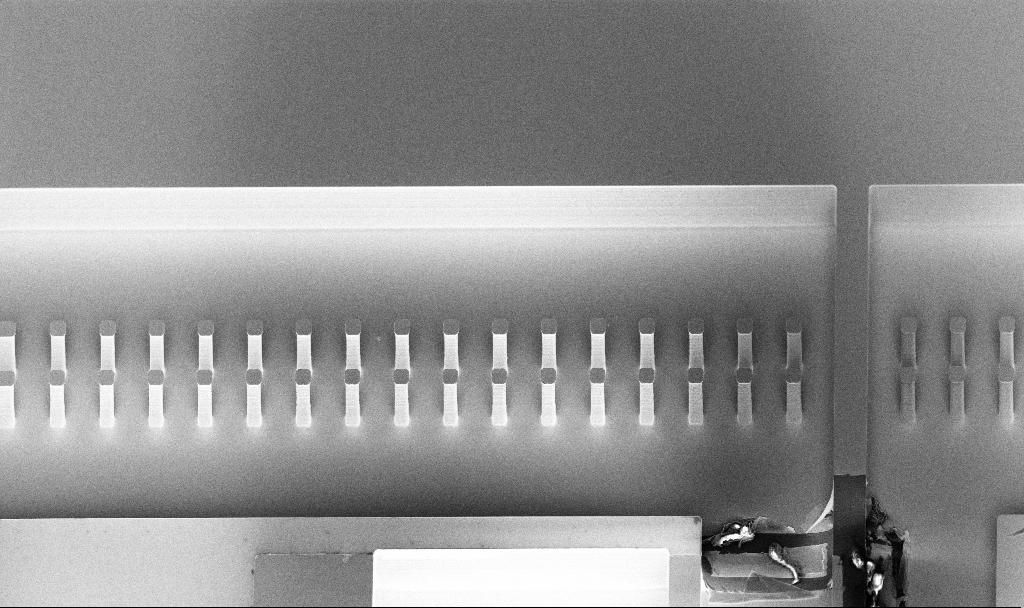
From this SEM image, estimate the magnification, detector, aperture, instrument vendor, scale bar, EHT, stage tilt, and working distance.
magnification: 0.6 K X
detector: InLens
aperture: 30 µm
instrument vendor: Zeiss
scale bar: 100000 nm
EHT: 5 kV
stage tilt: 45°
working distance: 8.2 mm